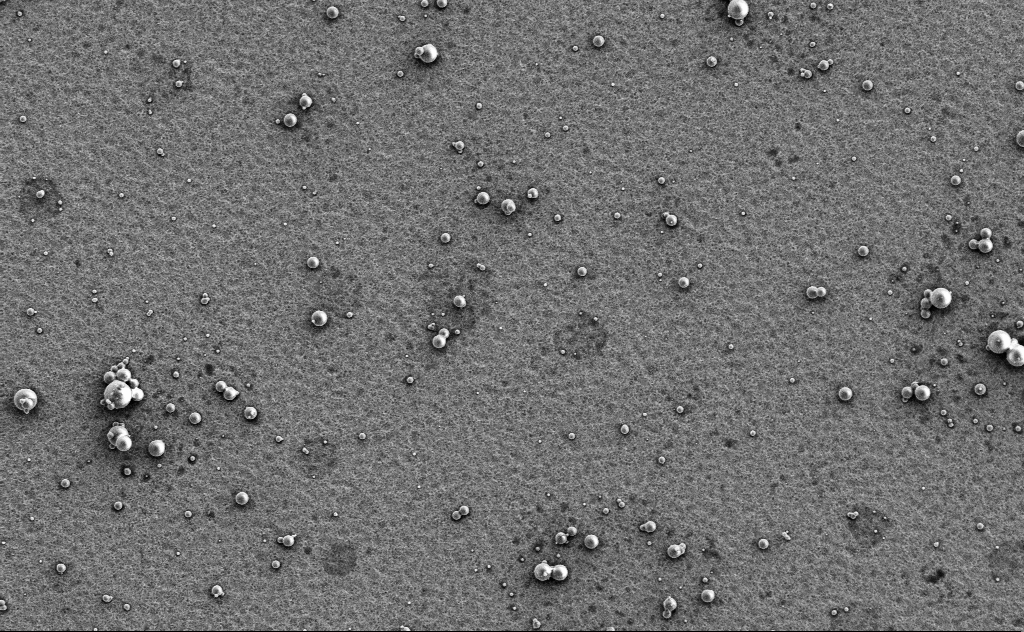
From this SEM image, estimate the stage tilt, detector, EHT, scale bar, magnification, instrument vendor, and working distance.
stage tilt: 0°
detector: SE2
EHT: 3 kV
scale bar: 10000 nm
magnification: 3.67 K X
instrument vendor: Zeiss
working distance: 13 mm